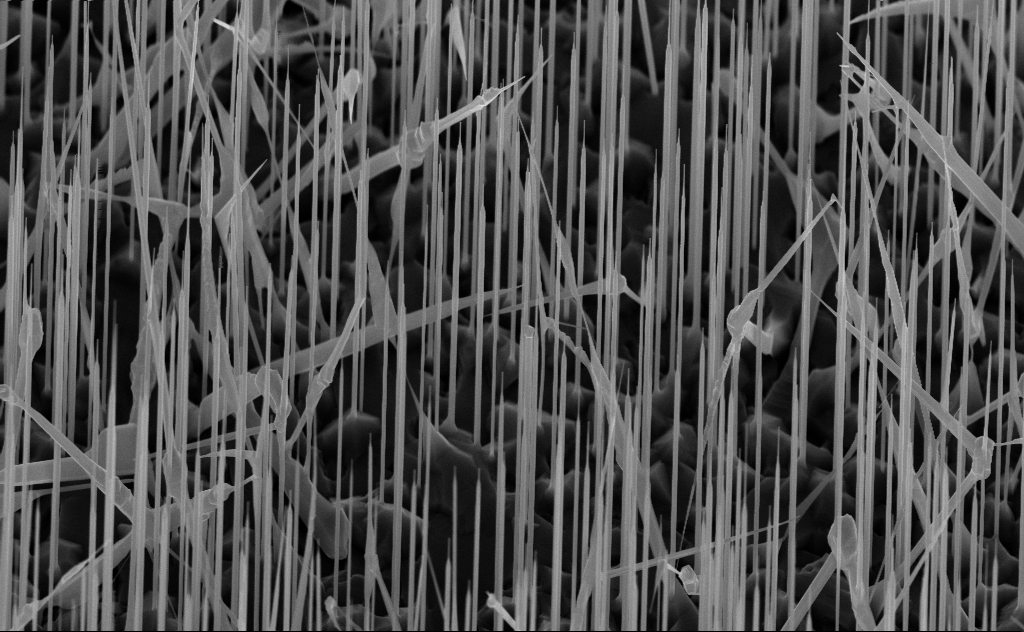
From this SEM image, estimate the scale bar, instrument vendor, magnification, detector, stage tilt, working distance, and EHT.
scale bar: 2000 nm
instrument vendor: Zeiss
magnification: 20 K X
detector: InLens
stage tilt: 44.9°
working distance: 7 mm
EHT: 10 kV